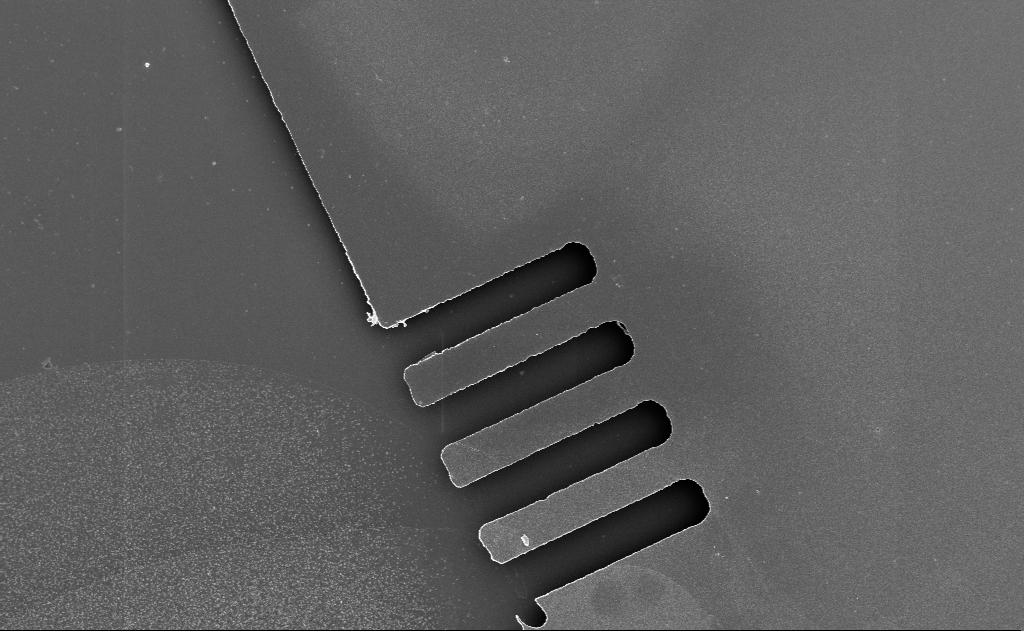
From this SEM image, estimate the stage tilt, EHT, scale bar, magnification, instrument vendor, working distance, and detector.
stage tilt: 1.3°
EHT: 1 kV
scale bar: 10000 nm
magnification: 3.23 K X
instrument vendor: Zeiss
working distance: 6 mm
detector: InLens